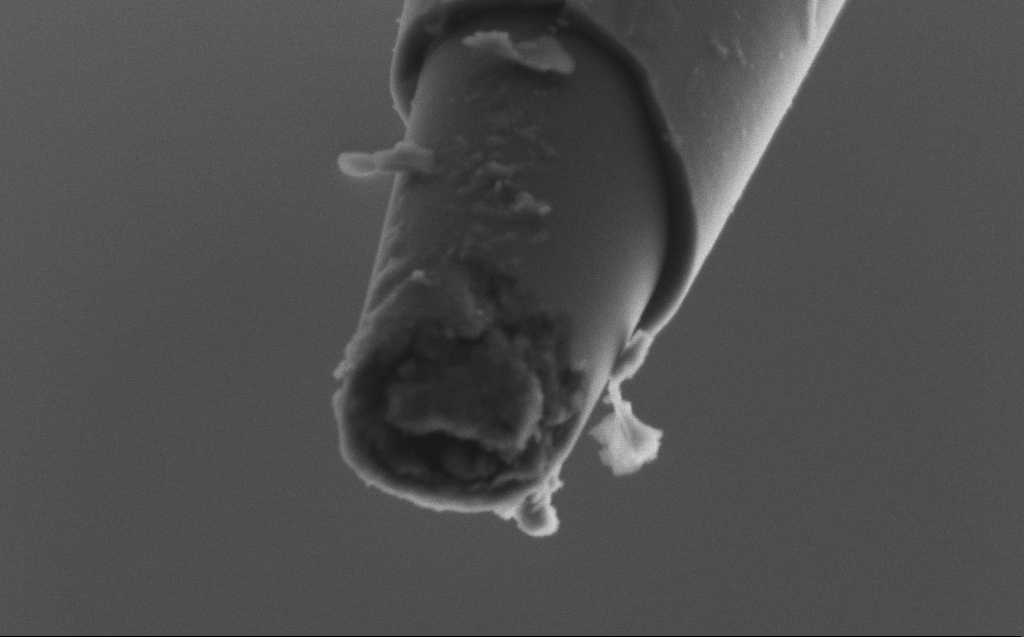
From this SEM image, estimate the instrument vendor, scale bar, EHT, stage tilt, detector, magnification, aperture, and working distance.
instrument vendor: Zeiss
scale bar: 200 nm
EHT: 2 kV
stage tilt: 45°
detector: SE2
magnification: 100 K X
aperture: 30 µm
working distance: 5 mm